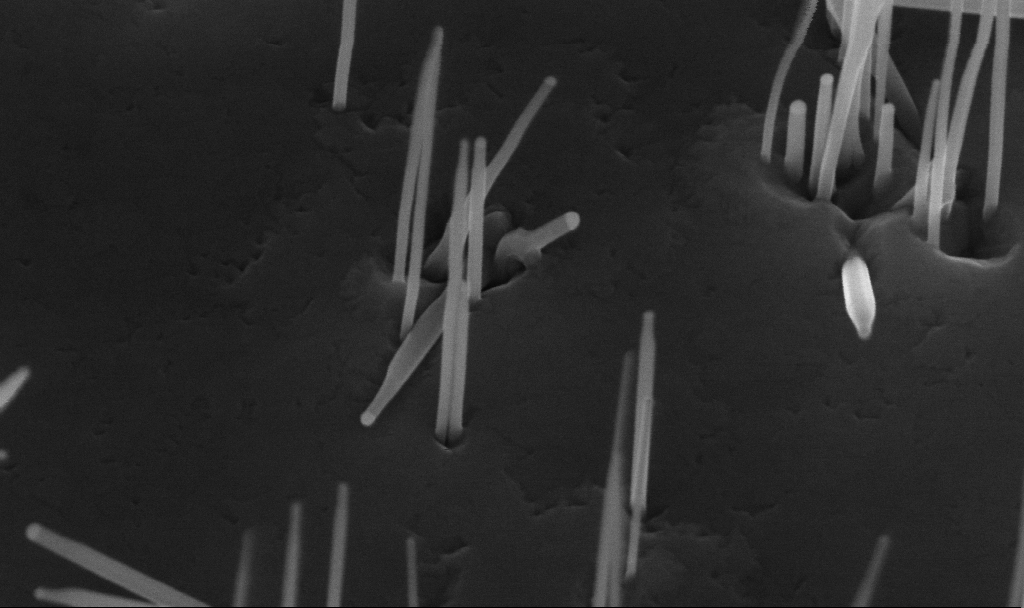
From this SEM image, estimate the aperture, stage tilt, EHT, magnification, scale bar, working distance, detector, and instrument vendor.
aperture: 30 µm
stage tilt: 45°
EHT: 10 kV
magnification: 155.83 K X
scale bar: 200 nm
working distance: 5.6 mm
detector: InLens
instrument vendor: Zeiss